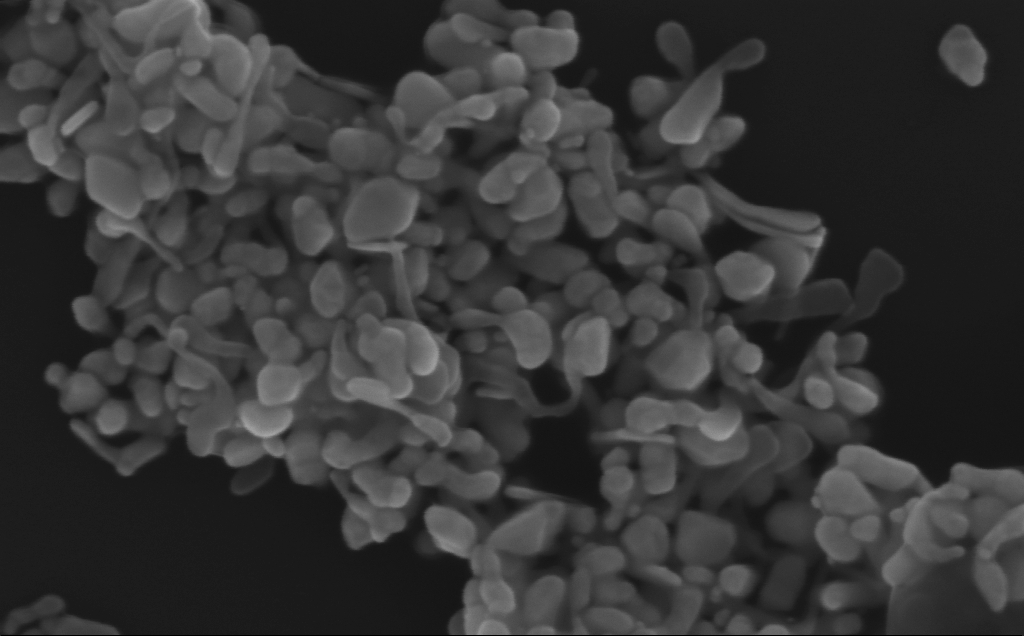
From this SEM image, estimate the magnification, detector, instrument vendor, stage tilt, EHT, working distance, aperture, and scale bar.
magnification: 268.67 K X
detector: InLens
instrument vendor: Zeiss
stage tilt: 0°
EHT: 10 kV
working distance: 3 mm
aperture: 30 µm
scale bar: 200 nm